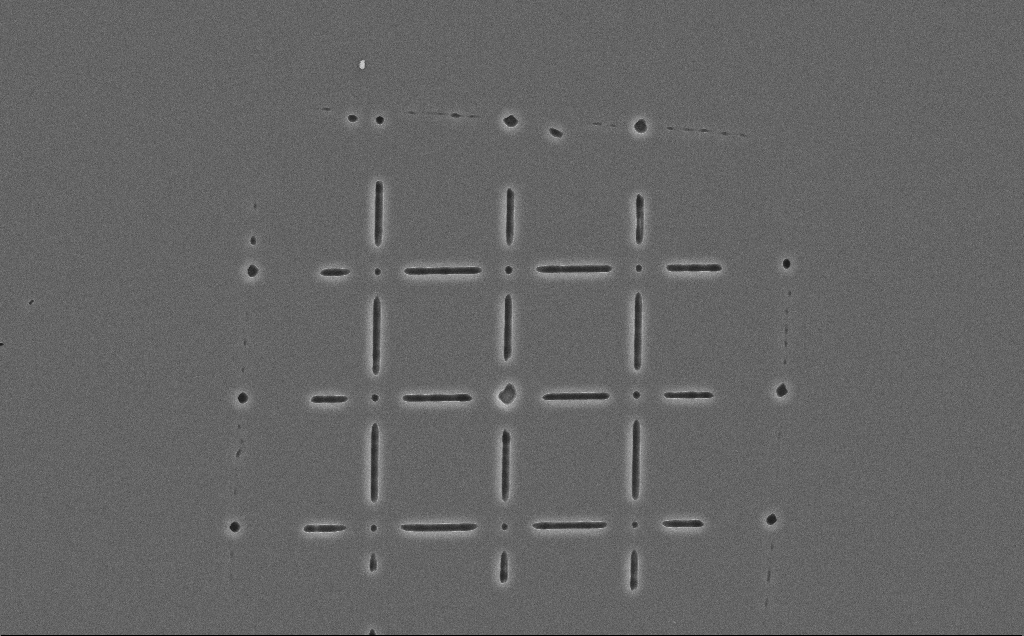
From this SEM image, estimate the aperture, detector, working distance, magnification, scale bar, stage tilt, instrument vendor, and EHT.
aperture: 30 µm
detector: SE2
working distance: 12 mm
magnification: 2.35 K X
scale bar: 10000 nm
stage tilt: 0°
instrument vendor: Zeiss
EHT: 10 kV